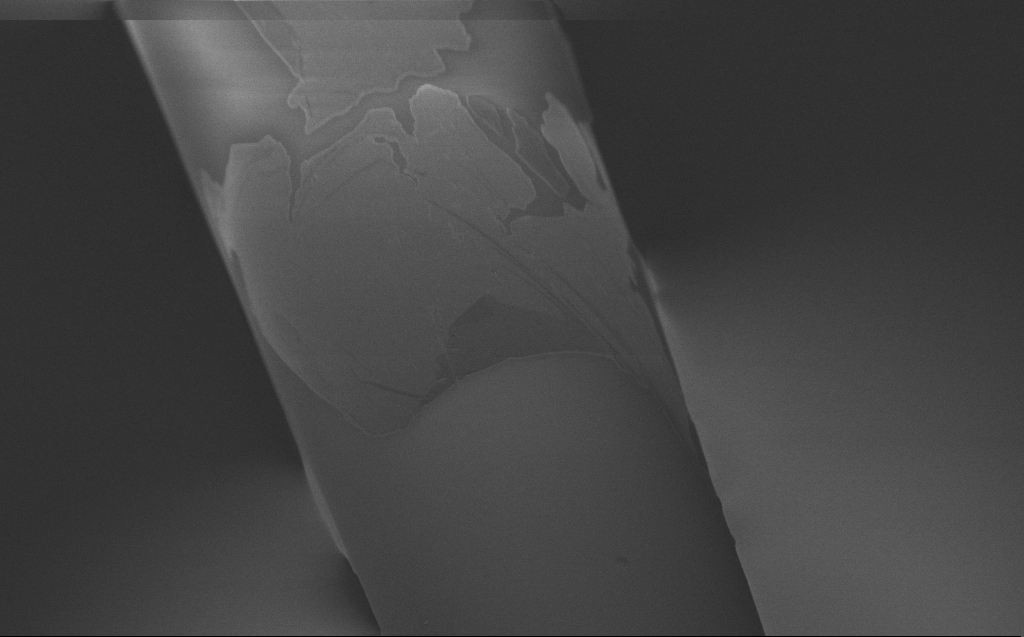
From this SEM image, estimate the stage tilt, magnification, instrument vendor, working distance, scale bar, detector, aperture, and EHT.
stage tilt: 45°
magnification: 5 K X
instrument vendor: Zeiss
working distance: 4 mm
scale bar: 10000 nm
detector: InLens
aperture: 30 µm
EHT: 1 kV